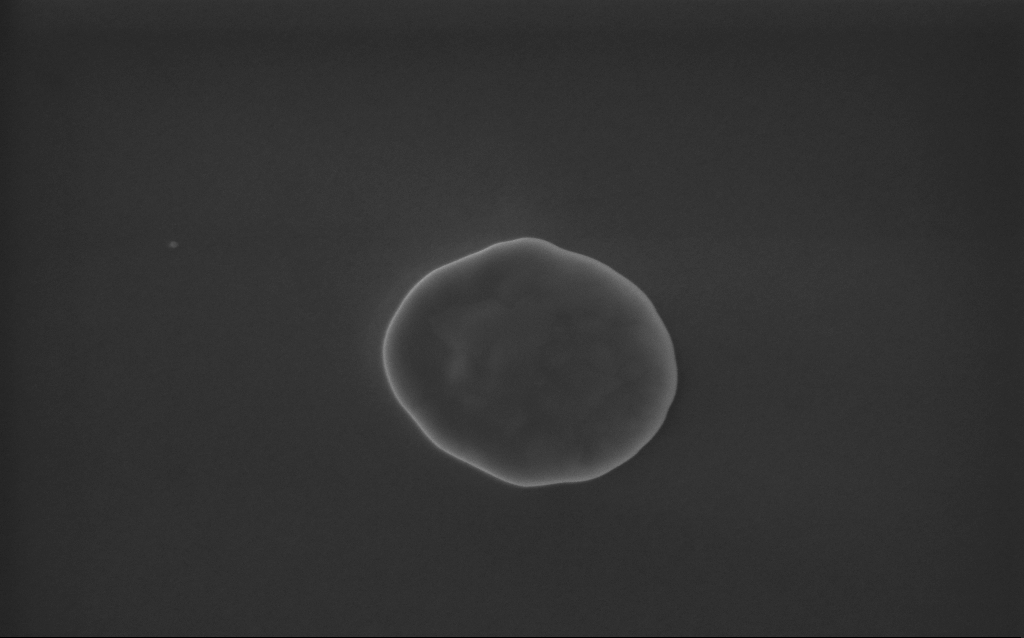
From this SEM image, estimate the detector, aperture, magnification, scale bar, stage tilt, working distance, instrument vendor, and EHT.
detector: InLens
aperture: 30 µm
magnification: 118 K X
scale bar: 200 nm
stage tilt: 0°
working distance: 3 mm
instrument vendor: Zeiss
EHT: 5 kV